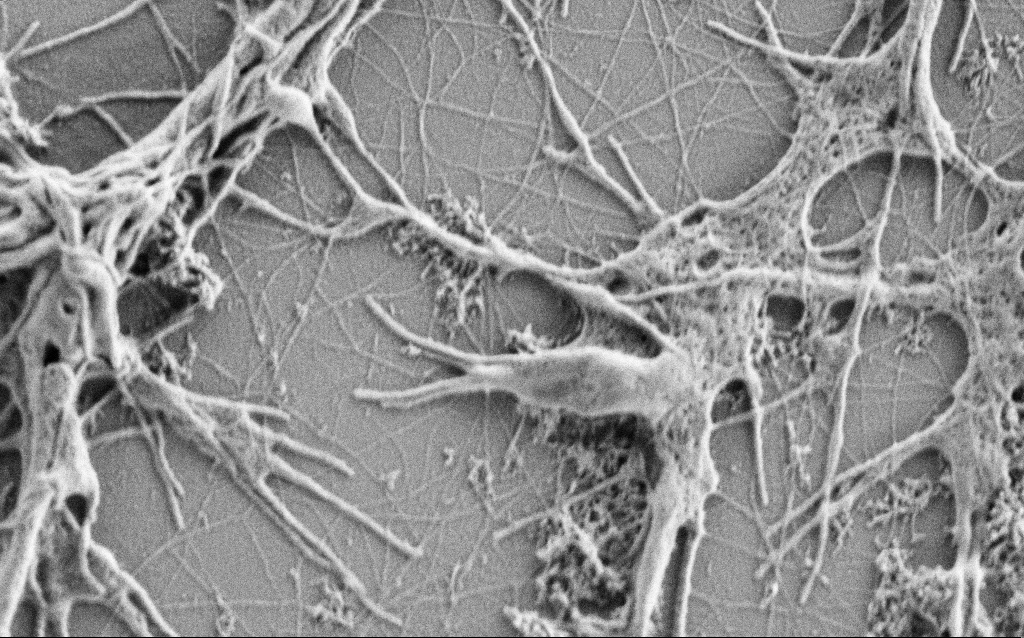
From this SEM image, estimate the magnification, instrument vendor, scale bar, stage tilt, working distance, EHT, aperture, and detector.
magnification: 25 K X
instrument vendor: Zeiss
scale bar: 2000 nm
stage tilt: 0°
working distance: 4 mm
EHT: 1 kV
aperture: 30 µm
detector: SE2